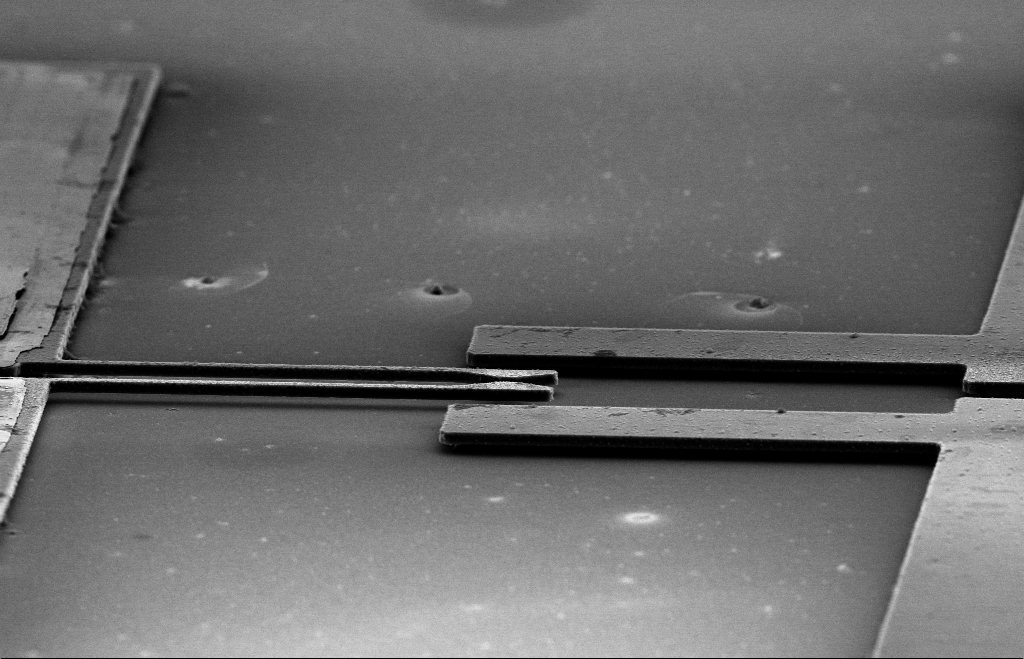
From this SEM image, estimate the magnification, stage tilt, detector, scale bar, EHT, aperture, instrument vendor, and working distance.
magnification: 1.86 K X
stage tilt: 70°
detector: SE2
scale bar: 10000 nm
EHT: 10 kV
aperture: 30 µm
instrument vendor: Zeiss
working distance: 11 mm